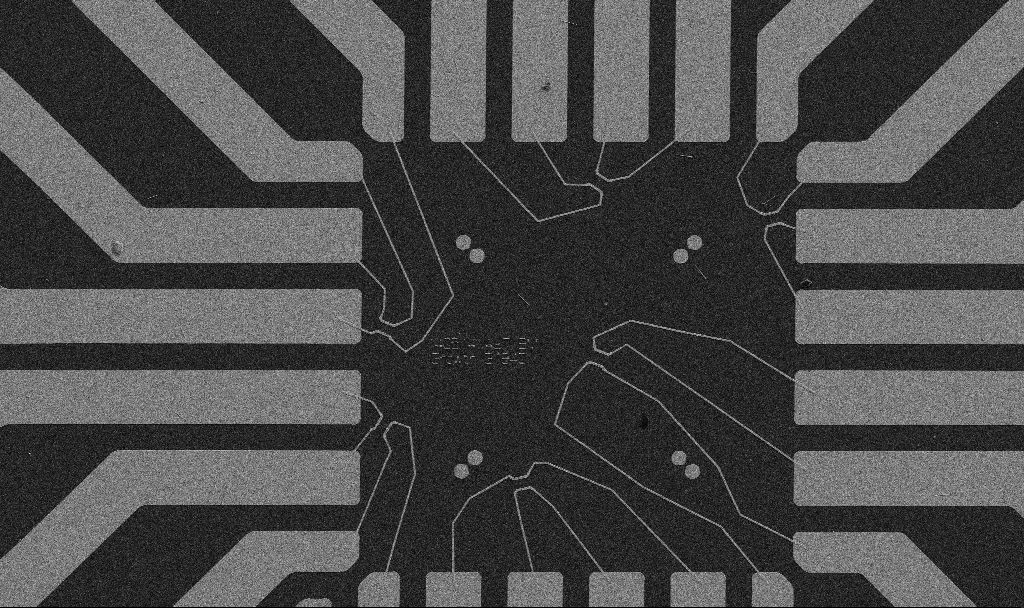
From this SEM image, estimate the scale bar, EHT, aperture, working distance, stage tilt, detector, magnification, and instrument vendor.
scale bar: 20000 nm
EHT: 5 kV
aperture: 30 µm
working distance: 10.7 mm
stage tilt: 0°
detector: SE2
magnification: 1 K X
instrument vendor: Zeiss